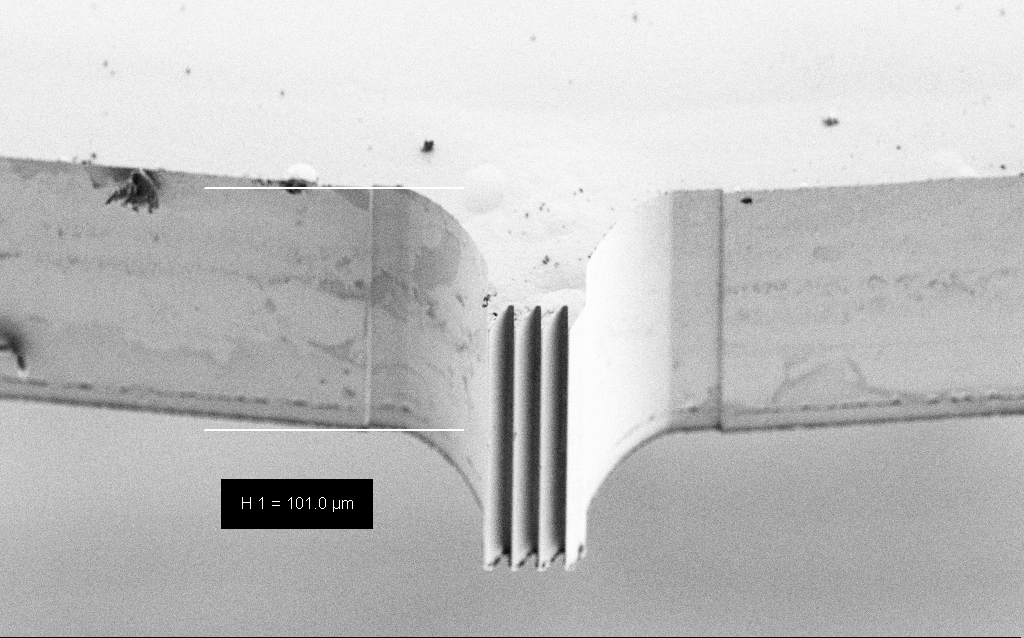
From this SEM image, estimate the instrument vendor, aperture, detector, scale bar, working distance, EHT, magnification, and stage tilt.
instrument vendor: Zeiss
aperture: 30 µm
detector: SE2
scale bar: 20000 nm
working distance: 4 mm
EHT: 3 kV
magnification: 0.88 K X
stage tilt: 45°